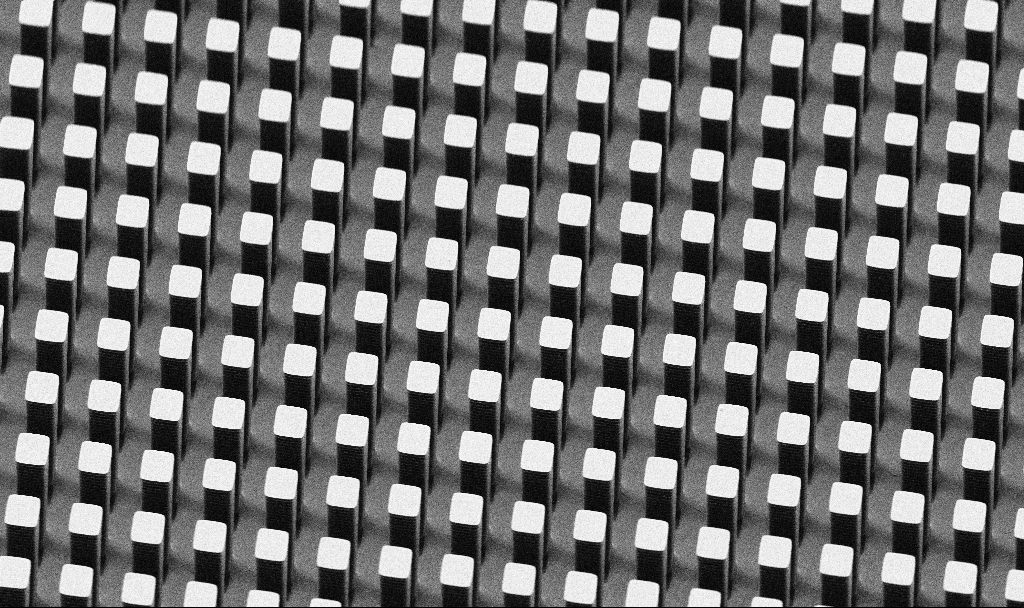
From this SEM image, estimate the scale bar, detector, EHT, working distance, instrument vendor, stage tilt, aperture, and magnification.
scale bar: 10000 nm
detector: SE2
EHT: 5 kV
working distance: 7.5 mm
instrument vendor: Zeiss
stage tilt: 45°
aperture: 30 µm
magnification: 2.88 K X